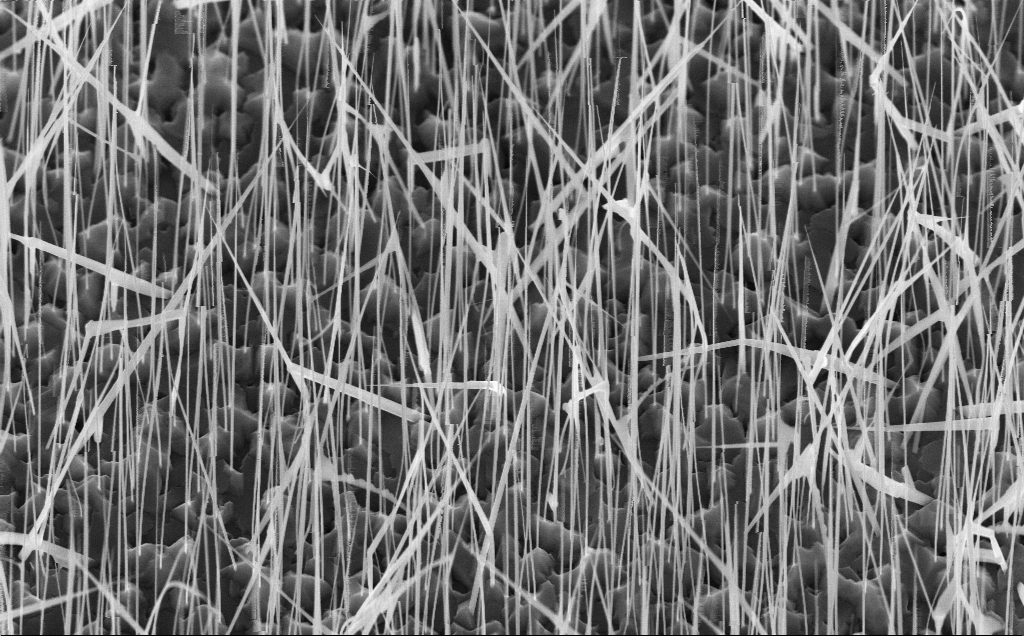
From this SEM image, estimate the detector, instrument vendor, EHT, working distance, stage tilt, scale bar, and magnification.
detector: InLens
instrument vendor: Zeiss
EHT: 10 kV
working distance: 7 mm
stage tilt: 45°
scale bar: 2000 nm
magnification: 23.41 K X